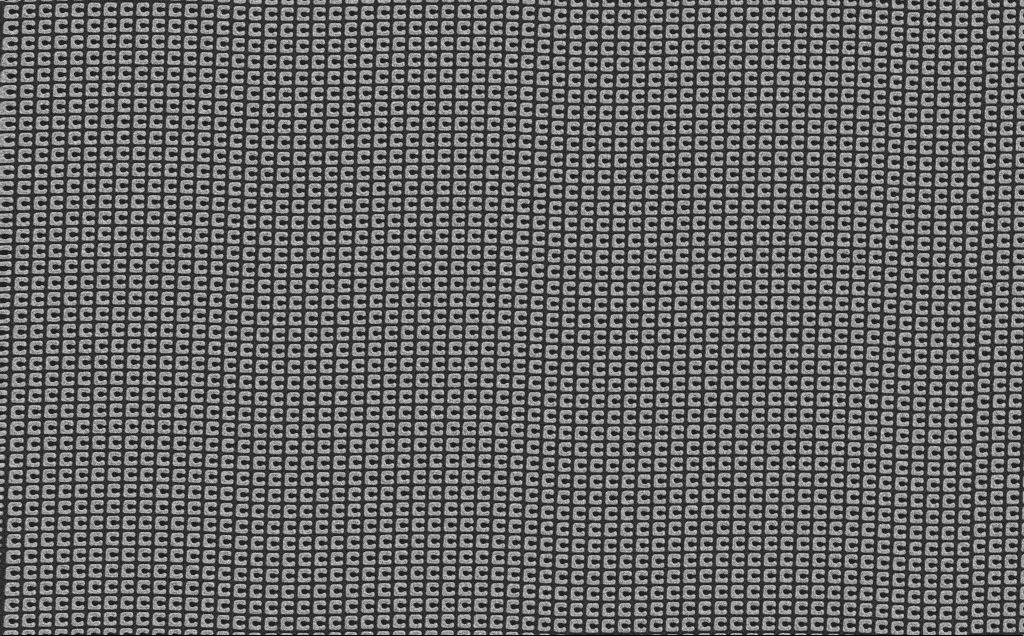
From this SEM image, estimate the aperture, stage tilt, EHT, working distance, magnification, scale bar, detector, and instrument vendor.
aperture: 30 µm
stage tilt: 0°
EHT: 5 kV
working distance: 2.3 mm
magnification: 12.82 K X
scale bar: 2000 nm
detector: SE2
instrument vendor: Zeiss